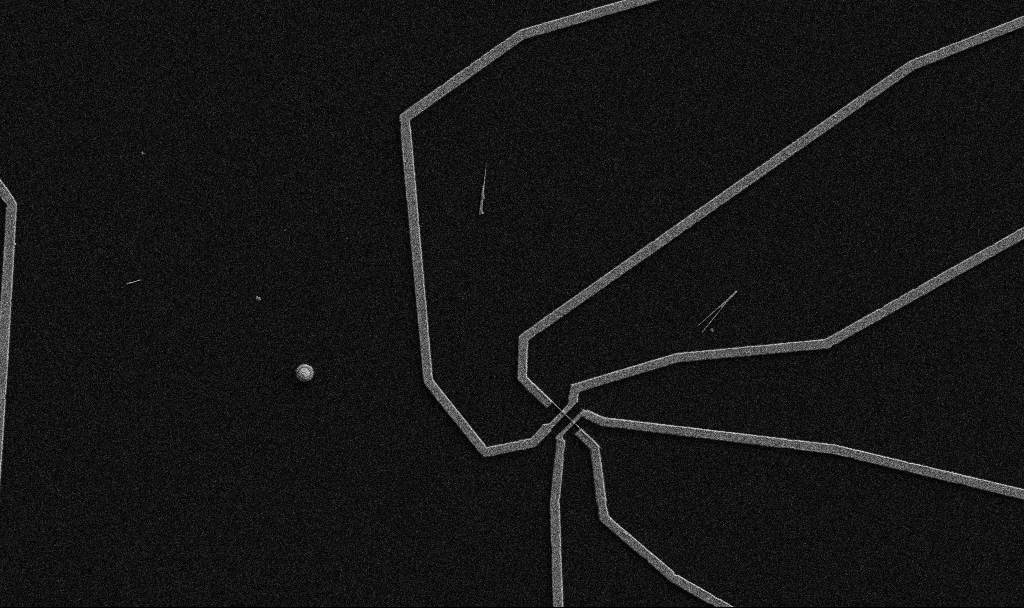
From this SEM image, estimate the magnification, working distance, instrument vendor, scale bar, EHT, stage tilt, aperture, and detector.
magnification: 5 K X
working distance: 10.7 mm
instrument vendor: Zeiss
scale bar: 10000 nm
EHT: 5 kV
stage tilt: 0°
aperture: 30 µm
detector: SE2